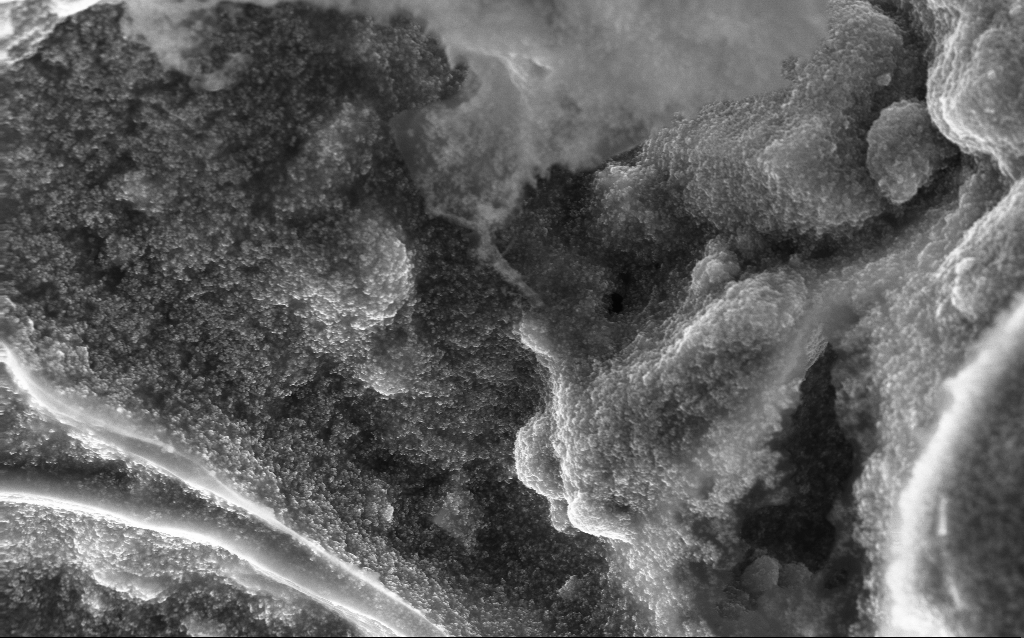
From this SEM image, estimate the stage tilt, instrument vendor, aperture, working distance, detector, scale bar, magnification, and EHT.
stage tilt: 0°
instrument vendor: Zeiss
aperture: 30 µm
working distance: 2.8 mm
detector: InLens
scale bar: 1000 nm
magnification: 15.33 K X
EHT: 10 kV